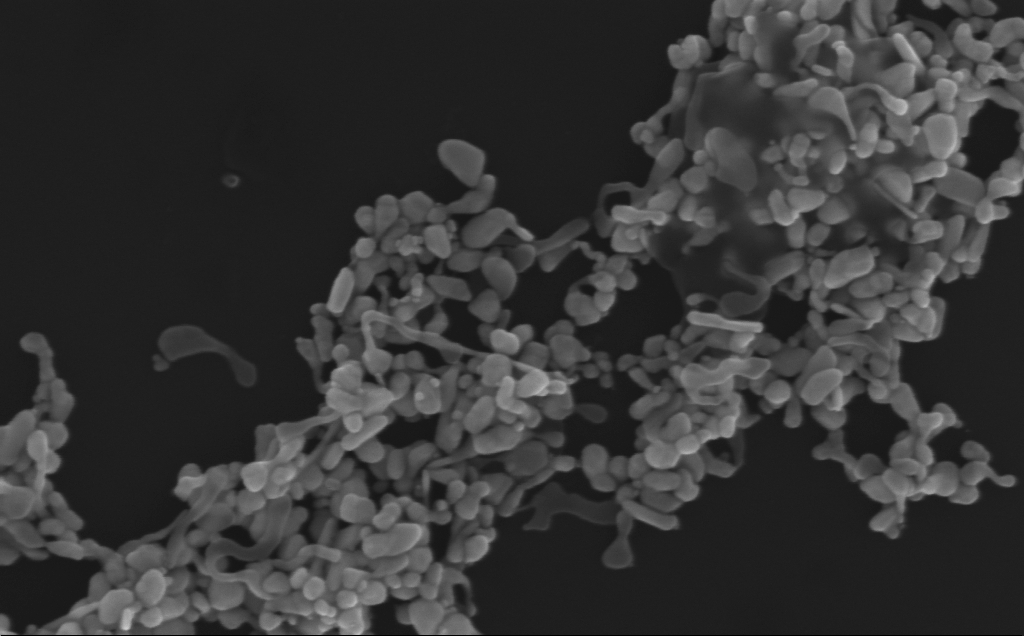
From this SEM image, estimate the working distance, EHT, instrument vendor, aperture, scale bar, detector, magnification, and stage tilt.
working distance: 3 mm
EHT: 10 kV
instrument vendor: Zeiss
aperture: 30 µm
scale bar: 200 nm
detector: InLens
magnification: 165.96 K X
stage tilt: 0°